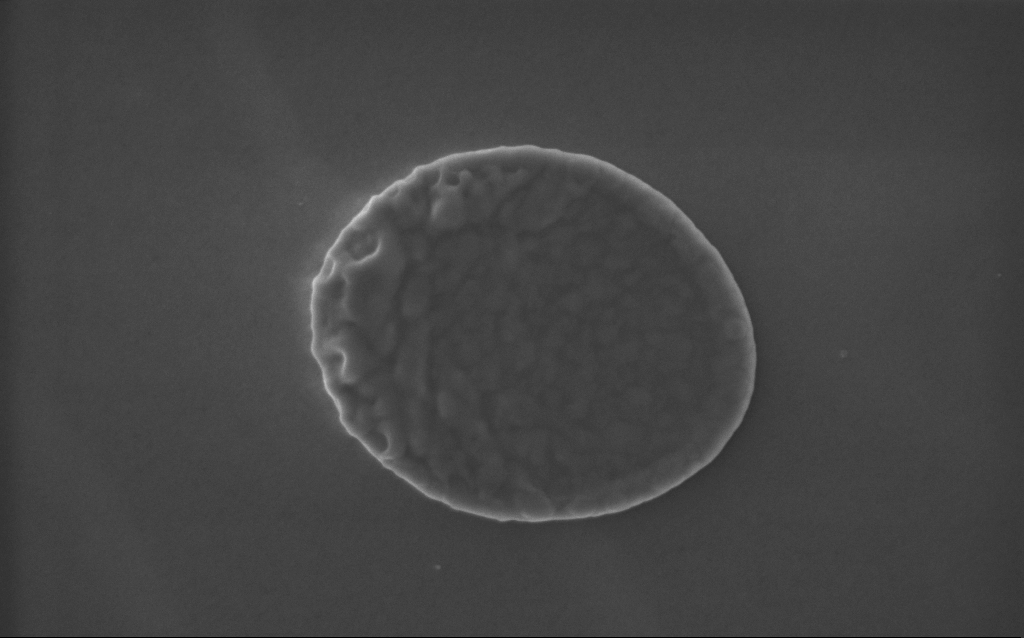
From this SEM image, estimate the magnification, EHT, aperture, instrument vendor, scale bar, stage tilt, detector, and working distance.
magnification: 91 K X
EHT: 5 kV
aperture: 30 µm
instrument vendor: Zeiss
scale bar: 200 nm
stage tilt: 0°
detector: InLens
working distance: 3 mm